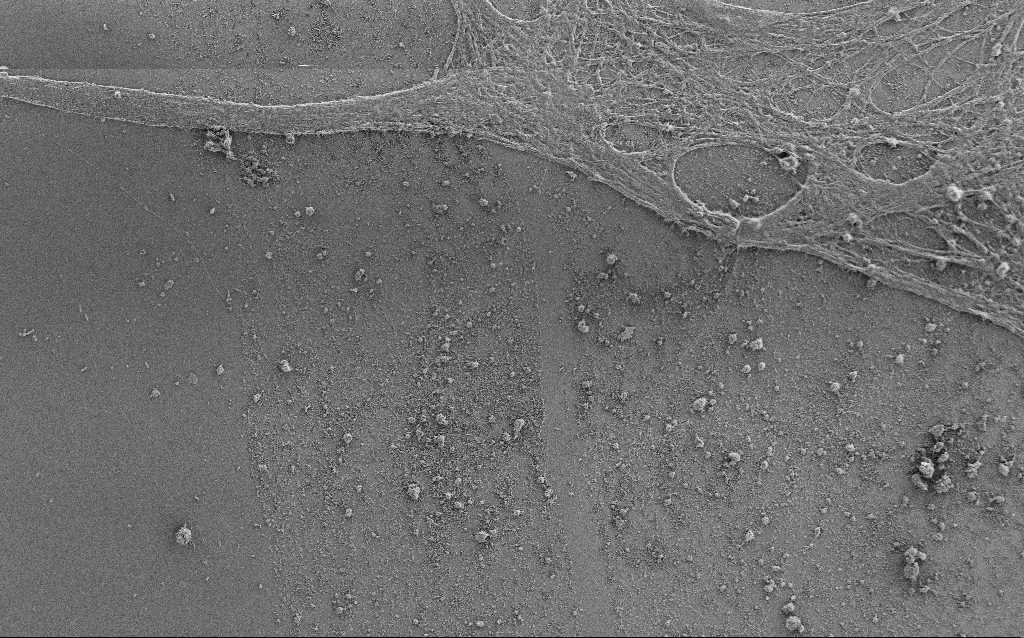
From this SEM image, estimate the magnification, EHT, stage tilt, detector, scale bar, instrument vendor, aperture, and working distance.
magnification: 1 K X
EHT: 0.9 kV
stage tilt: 0°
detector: SE2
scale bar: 20000 nm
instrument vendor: Zeiss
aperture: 30 µm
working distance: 4 mm